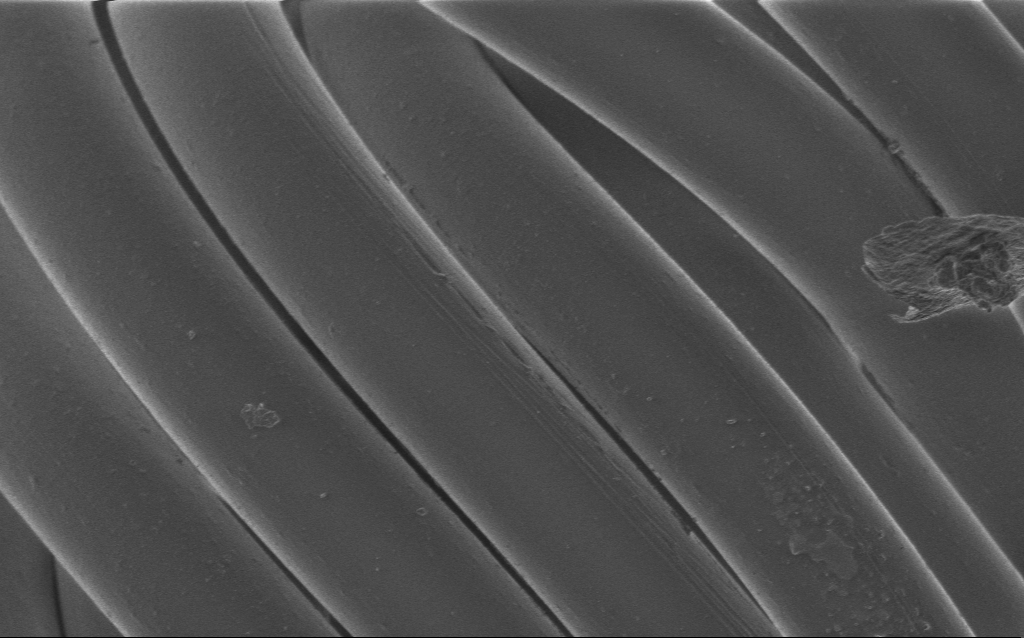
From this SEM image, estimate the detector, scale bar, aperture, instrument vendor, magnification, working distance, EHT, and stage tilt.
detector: InLens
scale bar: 20000 nm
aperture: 30 µm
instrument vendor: Zeiss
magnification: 2.49 K X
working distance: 4 mm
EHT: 1 kV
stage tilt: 0°